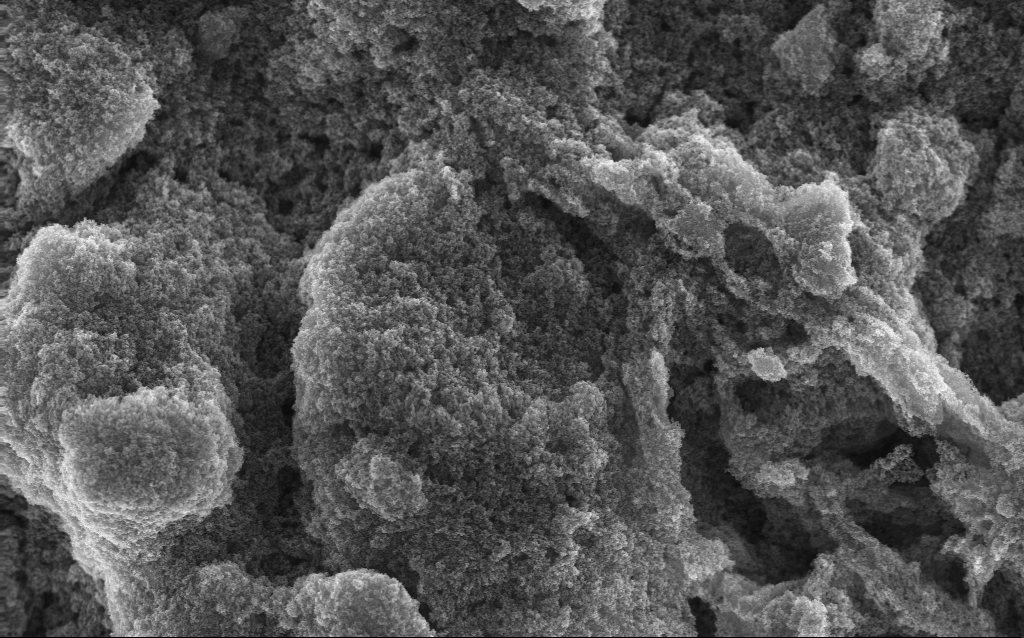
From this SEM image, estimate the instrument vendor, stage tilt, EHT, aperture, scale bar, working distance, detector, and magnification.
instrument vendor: Zeiss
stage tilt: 0°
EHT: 10 kV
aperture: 30 µm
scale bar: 1000 nm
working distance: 2.8 mm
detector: InLens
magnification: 20.87 K X